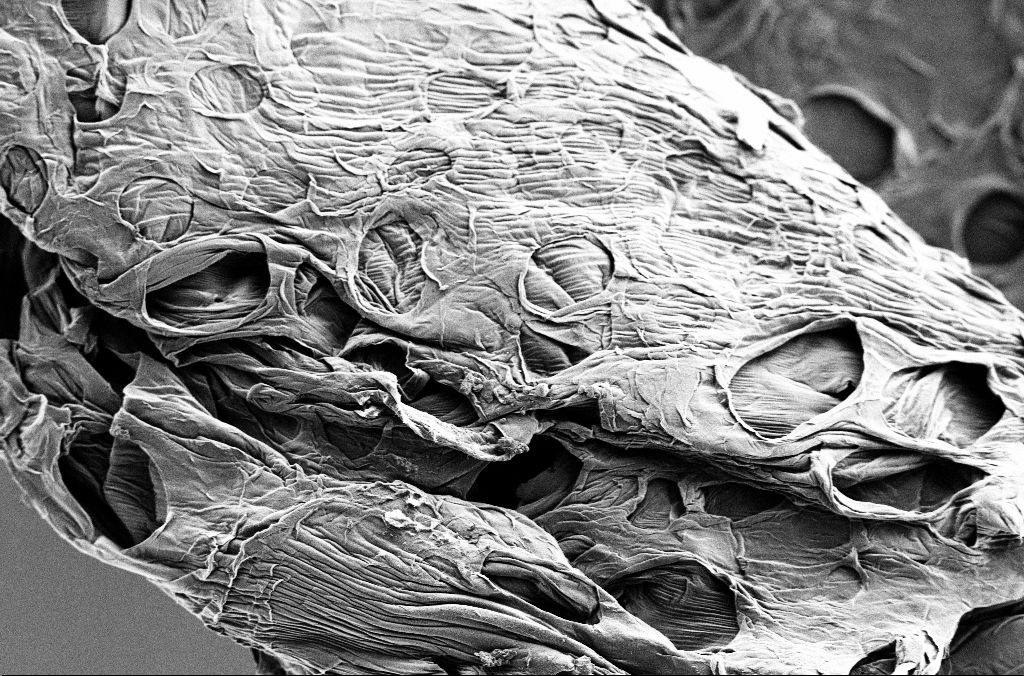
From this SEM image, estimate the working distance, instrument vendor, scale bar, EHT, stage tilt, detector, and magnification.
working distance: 5.4 mm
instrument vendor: Zeiss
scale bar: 20000 nm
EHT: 1.8 kV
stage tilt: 0°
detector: SE2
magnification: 1 K X